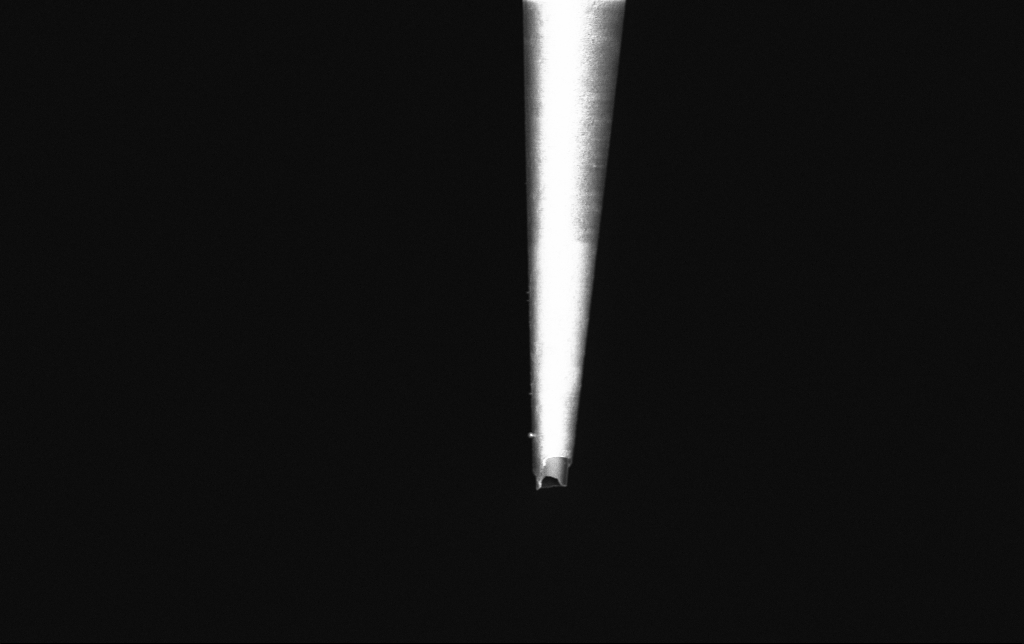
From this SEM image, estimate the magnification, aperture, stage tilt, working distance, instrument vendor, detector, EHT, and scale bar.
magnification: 5 K X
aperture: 30 µm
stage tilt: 0°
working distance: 6.1 mm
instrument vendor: Zeiss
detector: InLens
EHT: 2 kV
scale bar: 10000 nm